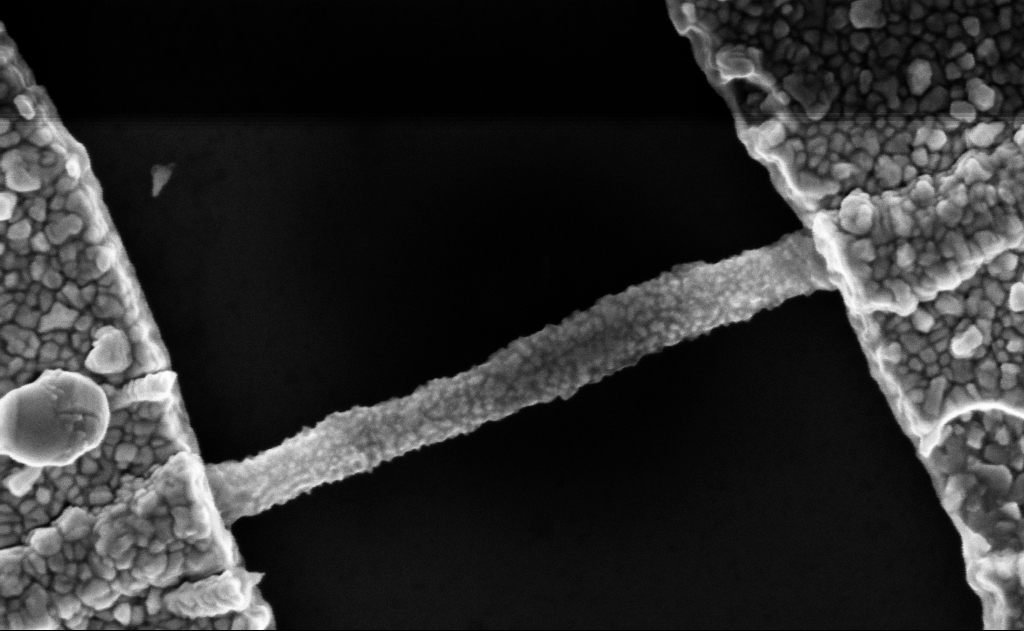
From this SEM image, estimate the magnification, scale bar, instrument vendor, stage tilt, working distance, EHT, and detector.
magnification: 128.92 K X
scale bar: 200 nm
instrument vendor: Zeiss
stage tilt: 0°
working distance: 7 mm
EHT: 5 kV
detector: InLens